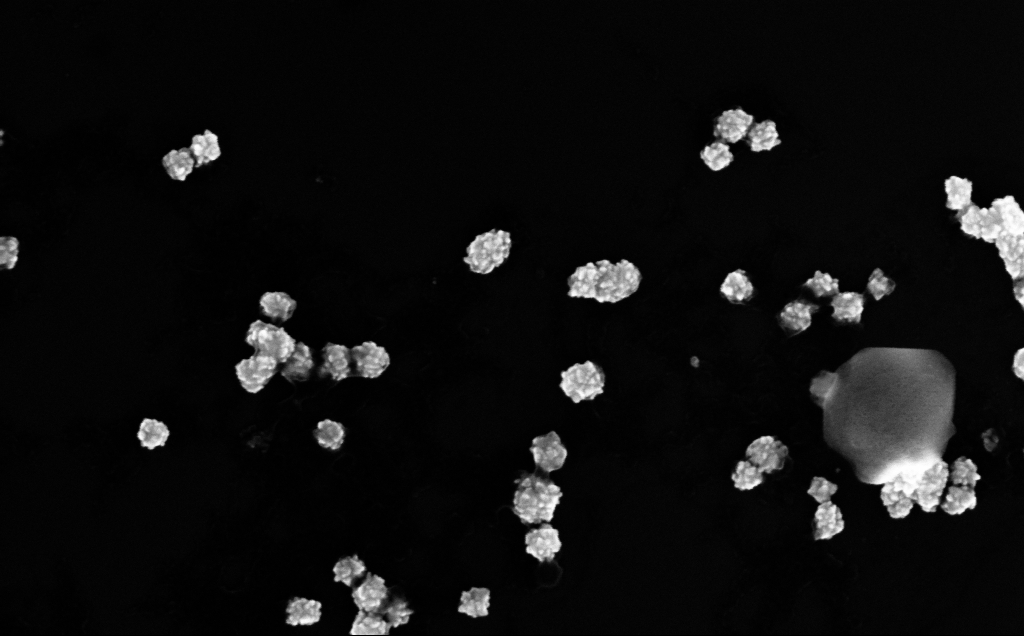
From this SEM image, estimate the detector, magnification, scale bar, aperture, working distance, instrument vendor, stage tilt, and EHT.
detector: InLens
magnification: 182.48 K X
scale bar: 200 nm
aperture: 30 µm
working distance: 4 mm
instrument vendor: Zeiss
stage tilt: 0°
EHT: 10 kV